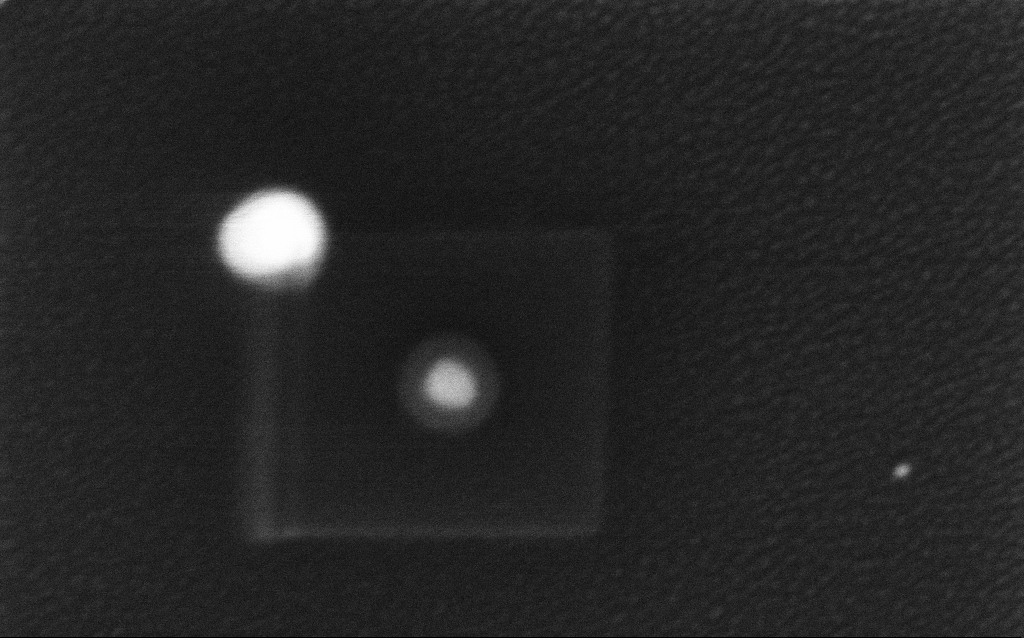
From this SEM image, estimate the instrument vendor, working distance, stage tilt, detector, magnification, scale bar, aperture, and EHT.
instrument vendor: Zeiss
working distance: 2.1 mm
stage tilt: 0°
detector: InLens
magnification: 298 K X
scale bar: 200 nm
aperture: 30 µm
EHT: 4 kV